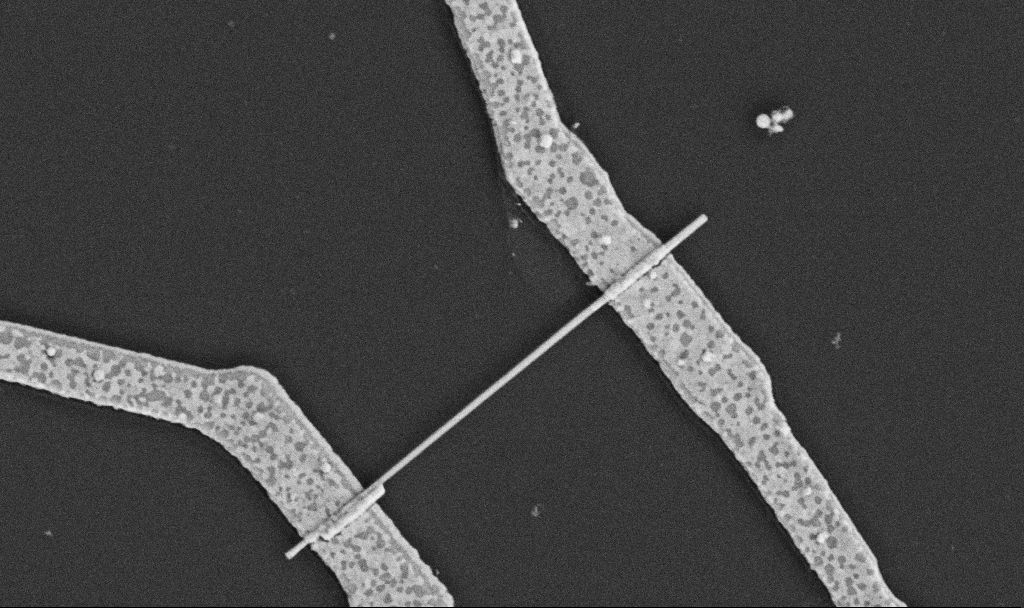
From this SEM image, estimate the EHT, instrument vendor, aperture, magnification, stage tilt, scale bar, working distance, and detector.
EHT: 5 kV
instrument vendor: Zeiss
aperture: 30 µm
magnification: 30 K X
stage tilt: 0°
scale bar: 1000 nm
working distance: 10.6 mm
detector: SE2